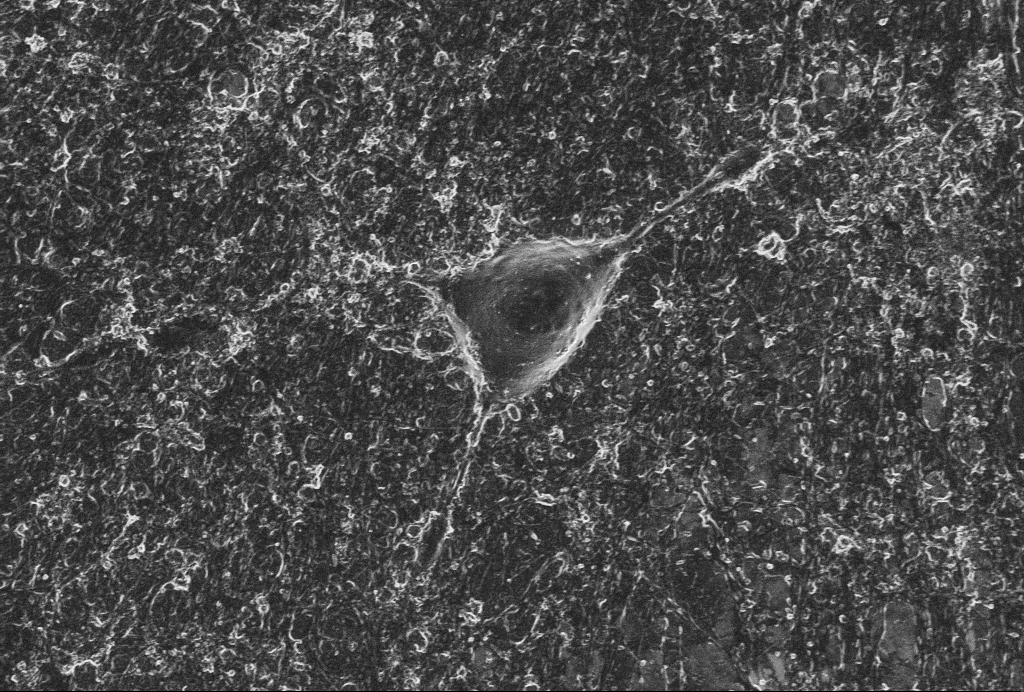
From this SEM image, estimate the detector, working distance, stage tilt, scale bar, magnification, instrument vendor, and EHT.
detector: InLens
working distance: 6 mm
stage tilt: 0°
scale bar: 10000 nm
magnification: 5 K X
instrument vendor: Zeiss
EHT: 2 kV